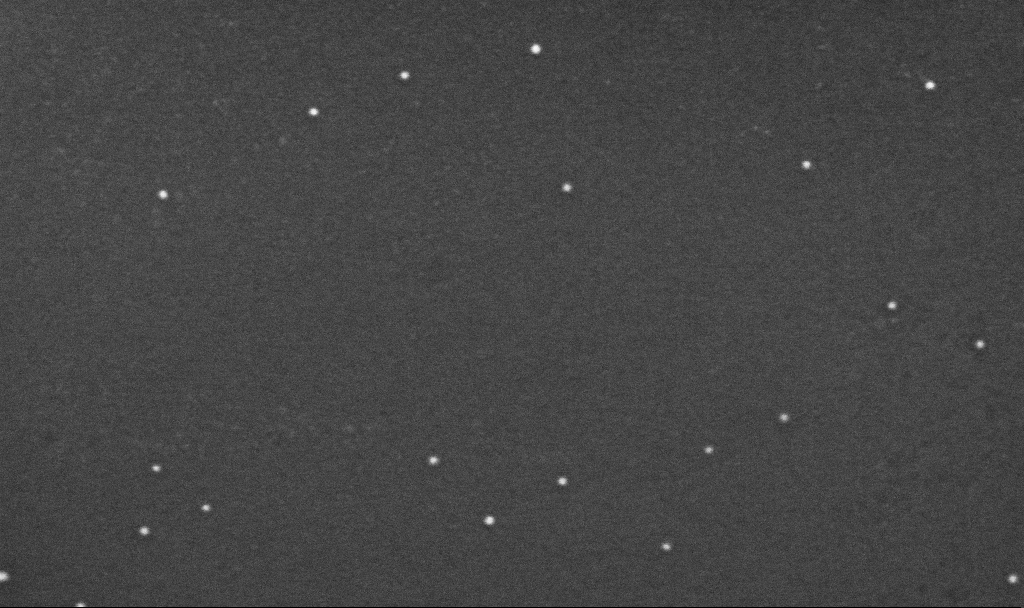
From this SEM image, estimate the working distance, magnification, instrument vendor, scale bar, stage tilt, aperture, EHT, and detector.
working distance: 3.2 mm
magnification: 250 K X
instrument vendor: Zeiss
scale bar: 100 nm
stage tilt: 0°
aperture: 30 µm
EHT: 10 kV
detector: InLens